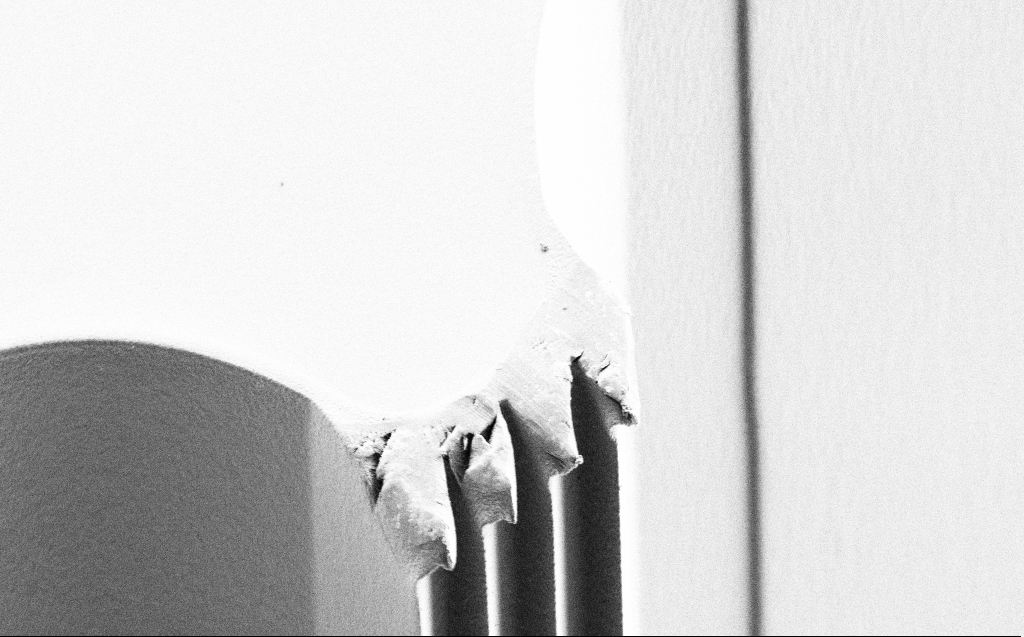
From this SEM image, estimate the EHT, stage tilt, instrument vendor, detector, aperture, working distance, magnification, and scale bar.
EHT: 1 kV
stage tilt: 44.6°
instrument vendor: Zeiss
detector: SE2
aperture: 30 µm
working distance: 5 mm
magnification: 2.62 K X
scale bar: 10000 nm